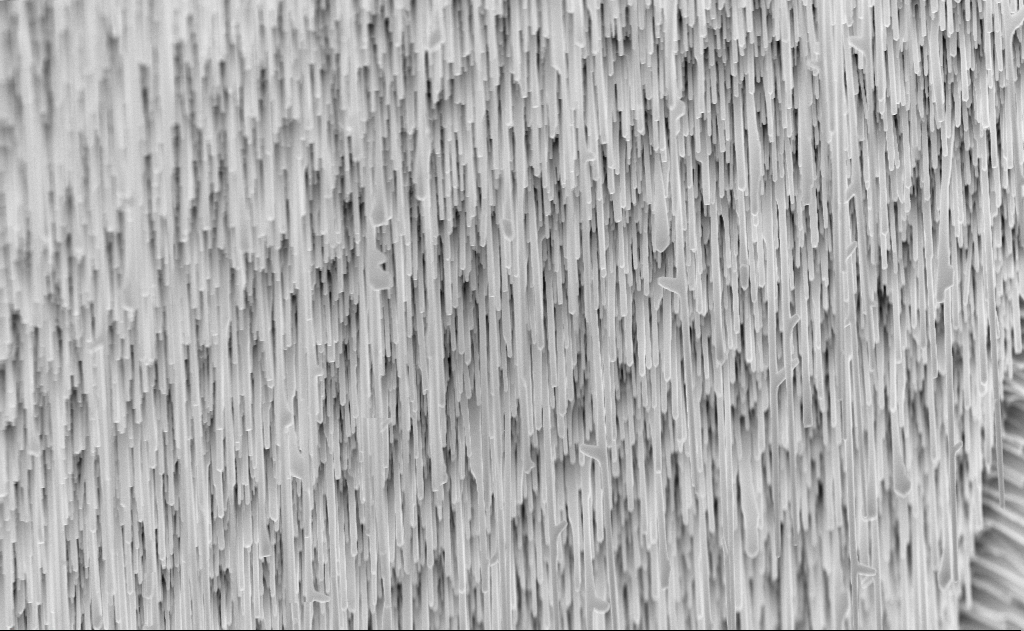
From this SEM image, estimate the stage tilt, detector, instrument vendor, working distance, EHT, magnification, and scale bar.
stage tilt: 0°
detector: InLens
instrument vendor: Zeiss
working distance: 6 mm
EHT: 10 kV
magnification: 20 K X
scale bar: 2000 nm